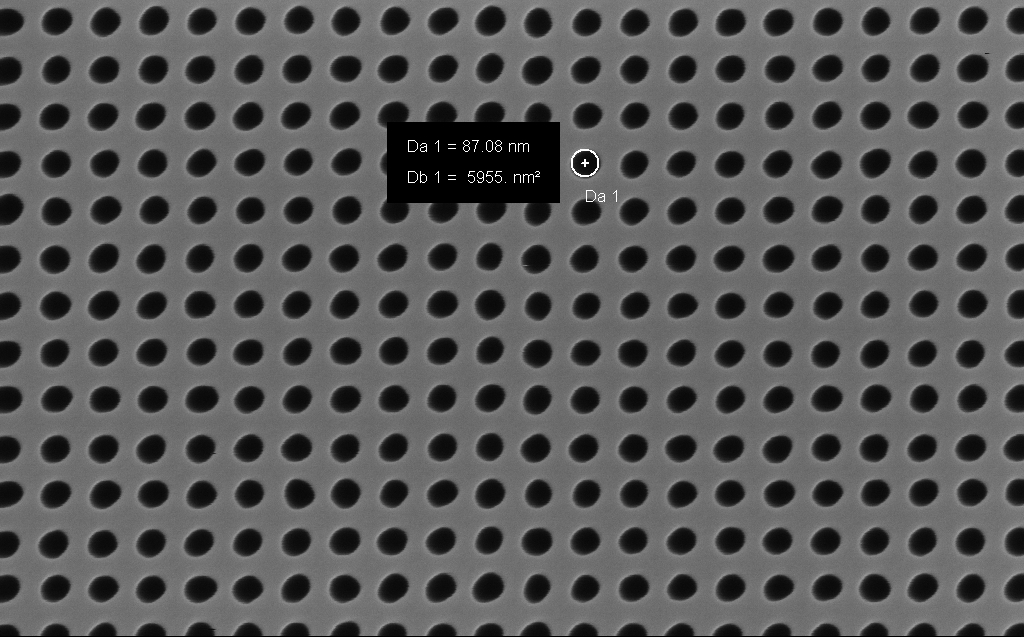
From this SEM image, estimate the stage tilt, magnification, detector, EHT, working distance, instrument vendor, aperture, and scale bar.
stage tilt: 0°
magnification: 118.07 K X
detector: InLens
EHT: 5 kV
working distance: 7 mm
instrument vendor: Zeiss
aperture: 30 µm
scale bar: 200 nm